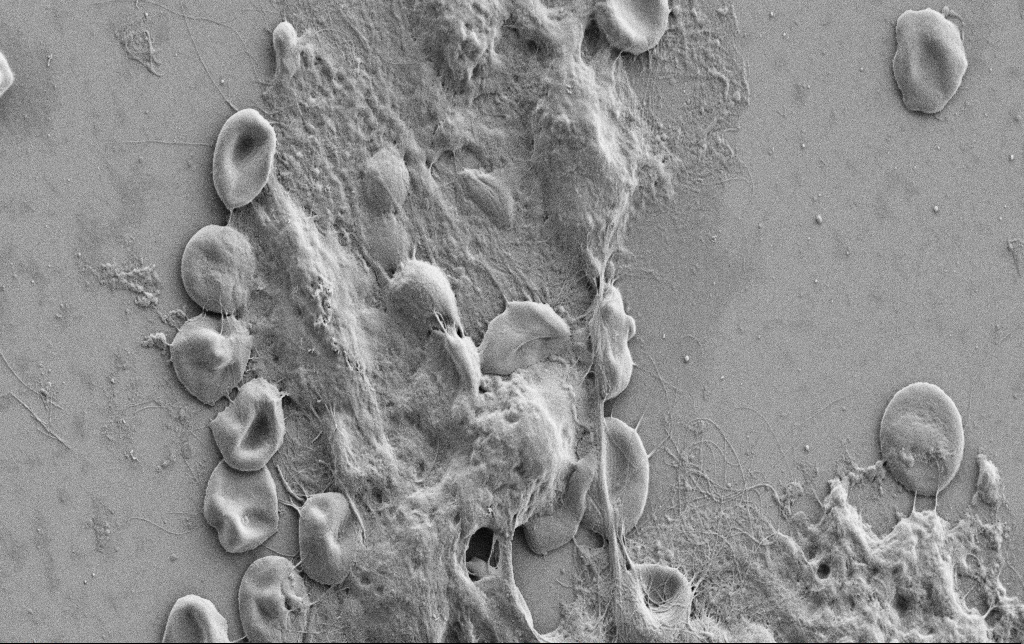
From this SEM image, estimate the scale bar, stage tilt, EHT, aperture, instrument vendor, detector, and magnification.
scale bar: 10000 nm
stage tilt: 0°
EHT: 2 kV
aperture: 30 µm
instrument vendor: Zeiss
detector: SE2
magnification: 5 K X